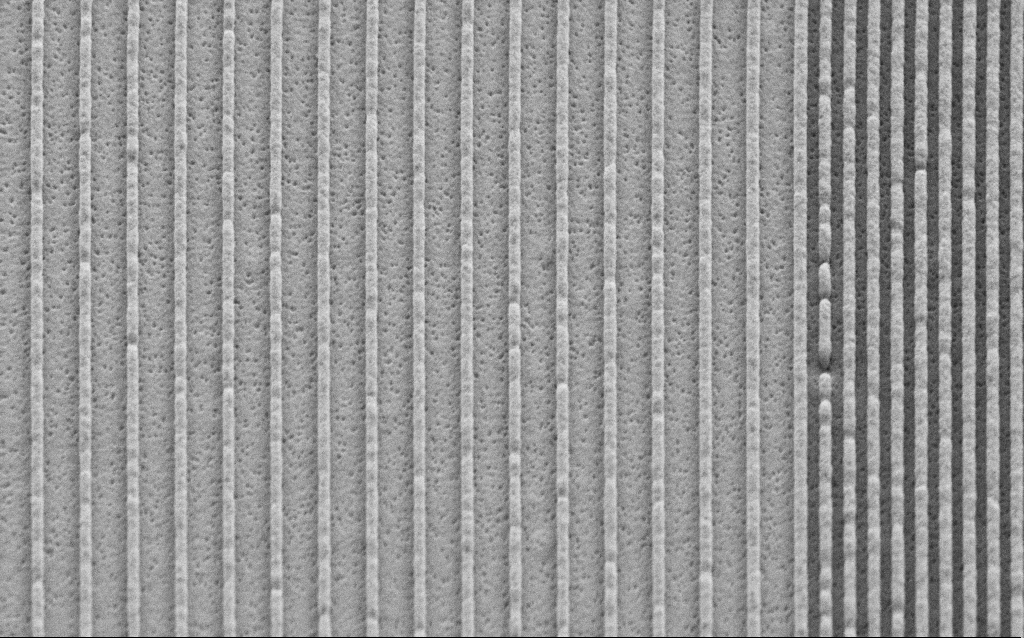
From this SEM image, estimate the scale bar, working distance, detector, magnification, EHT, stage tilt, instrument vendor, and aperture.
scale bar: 1000 nm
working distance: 6.6 mm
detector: SE2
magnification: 42.97 K X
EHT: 3 kV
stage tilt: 45°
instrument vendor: Zeiss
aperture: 30 µm